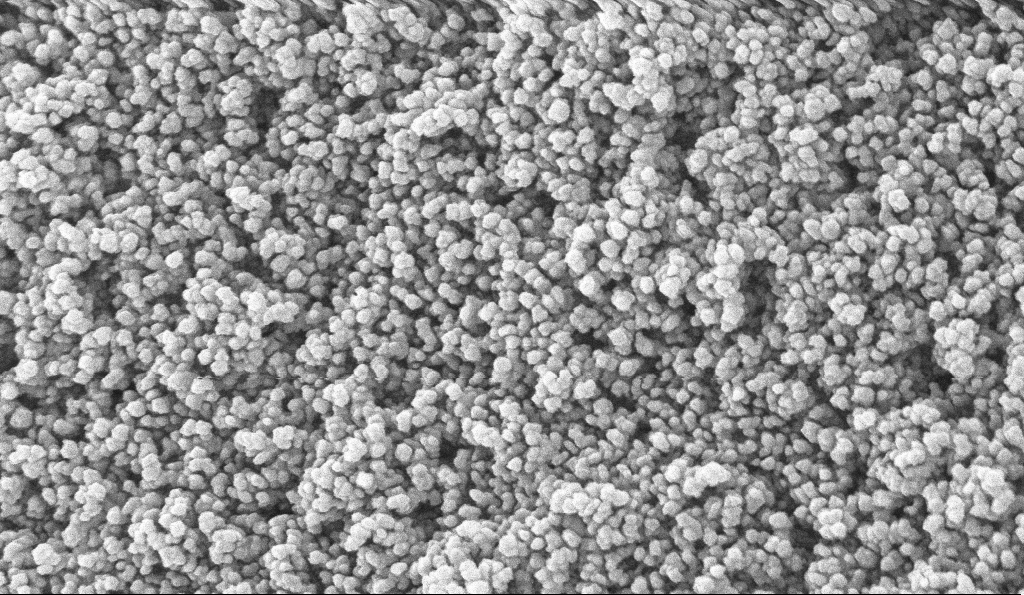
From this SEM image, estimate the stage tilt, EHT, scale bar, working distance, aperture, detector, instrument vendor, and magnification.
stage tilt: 0°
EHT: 10 kV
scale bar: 100 nm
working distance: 5.1 mm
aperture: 30 µm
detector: InLens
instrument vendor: Zeiss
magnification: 135 K X